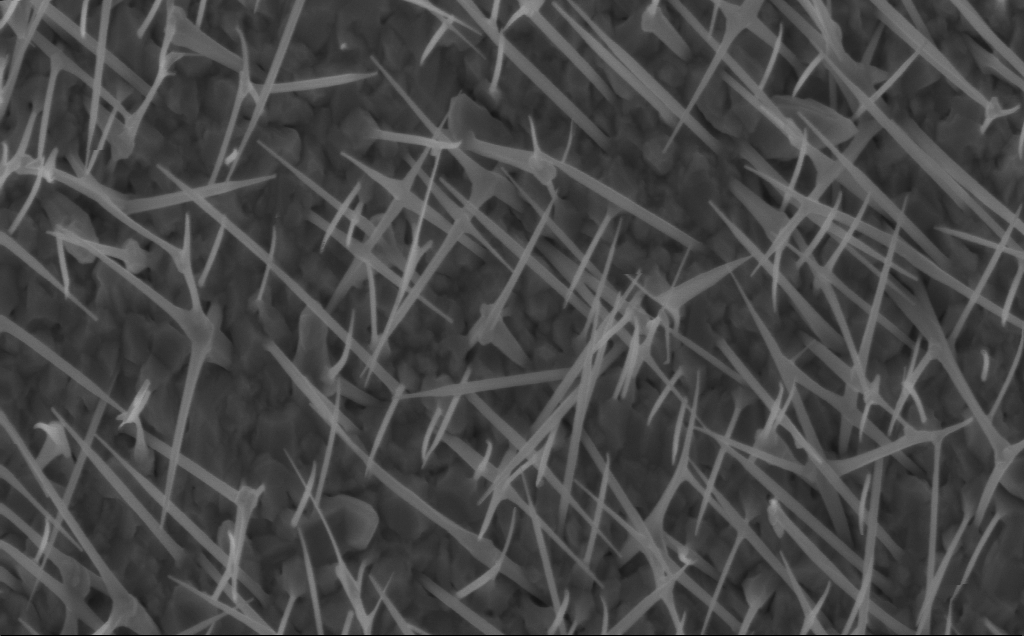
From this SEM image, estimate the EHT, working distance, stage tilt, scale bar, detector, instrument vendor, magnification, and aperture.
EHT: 5 kV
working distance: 5 mm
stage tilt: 0°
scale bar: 1000 nm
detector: InLens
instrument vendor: Zeiss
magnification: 40 K X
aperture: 30 µm